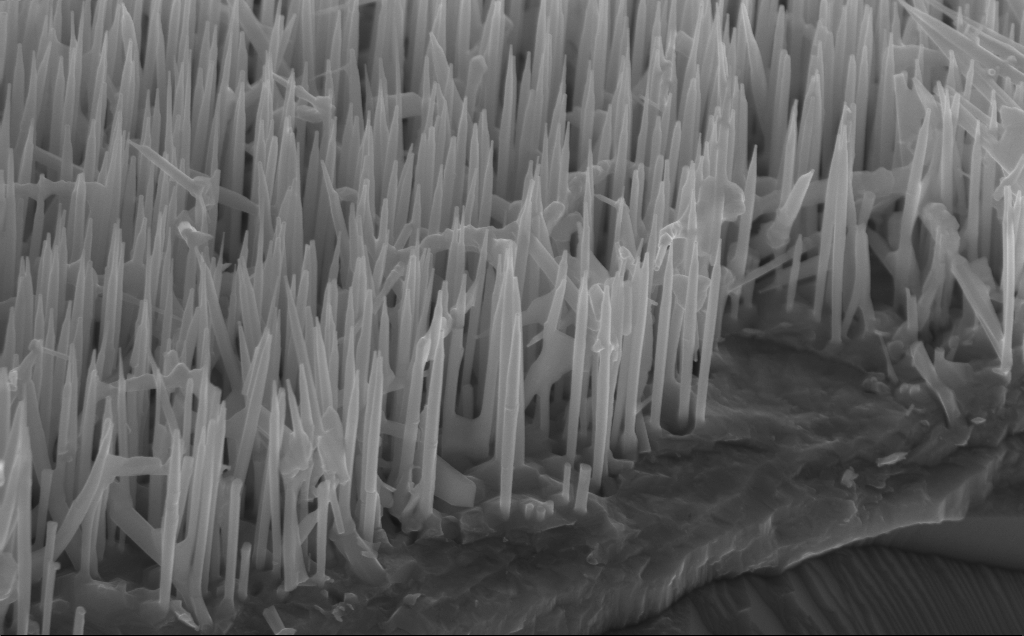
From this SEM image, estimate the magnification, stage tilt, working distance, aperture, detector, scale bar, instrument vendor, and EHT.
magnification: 40 K X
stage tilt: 45°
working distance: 5 mm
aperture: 30 µm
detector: InLens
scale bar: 1000 nm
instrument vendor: Zeiss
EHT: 12 kV